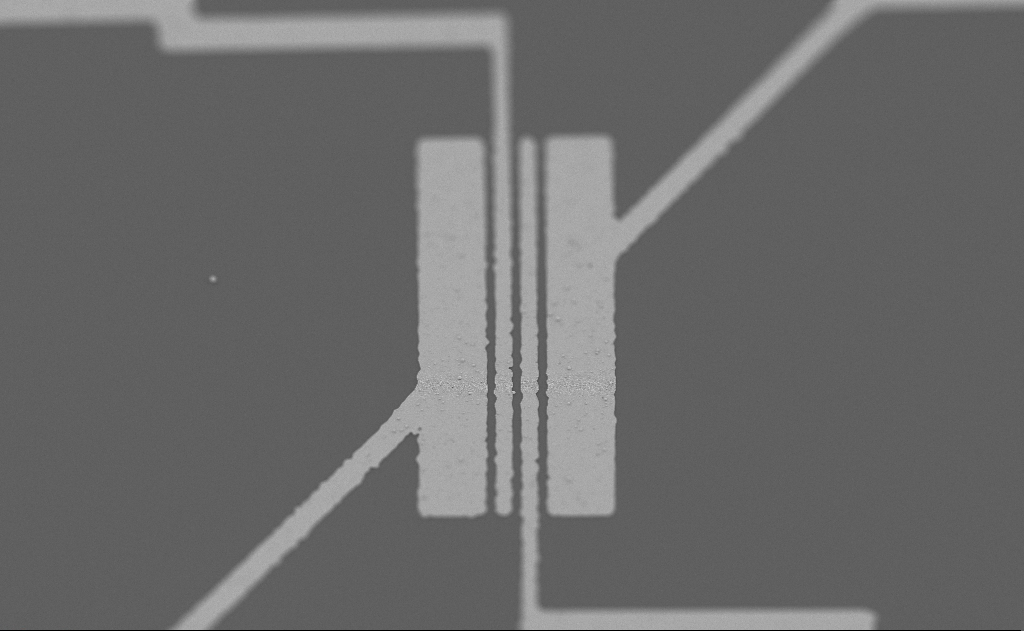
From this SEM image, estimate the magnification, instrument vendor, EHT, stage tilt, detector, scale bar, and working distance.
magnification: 2.38 K X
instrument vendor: Zeiss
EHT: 5 kV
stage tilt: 0°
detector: SE2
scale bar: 10000 nm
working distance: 10 mm